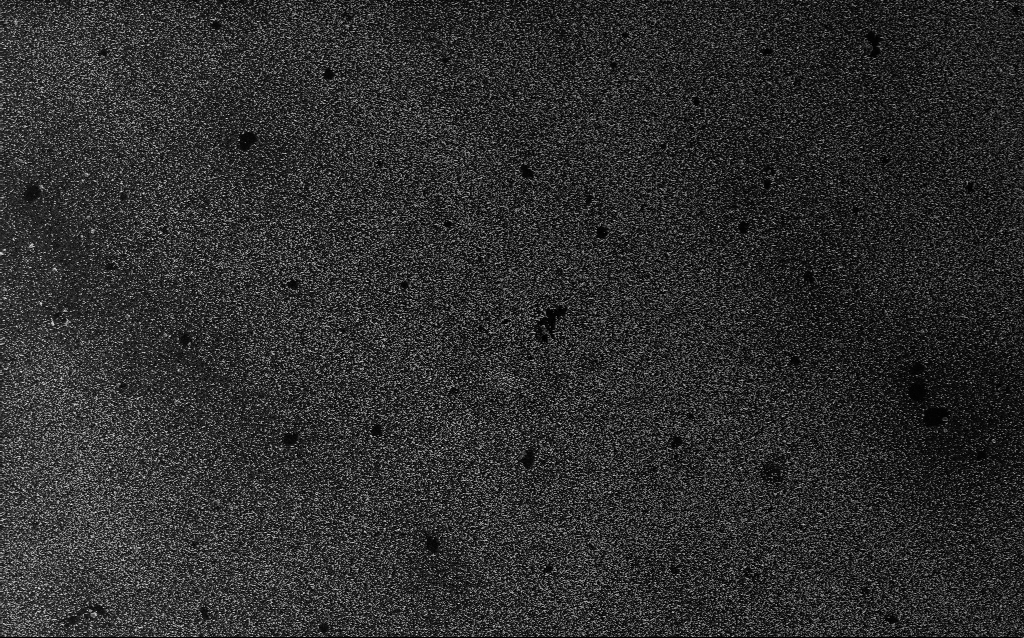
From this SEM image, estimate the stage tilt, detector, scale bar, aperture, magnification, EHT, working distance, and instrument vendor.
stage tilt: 0°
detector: InLens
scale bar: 10000 nm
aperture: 30 µm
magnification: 2 K X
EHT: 5 kV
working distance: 2.1 mm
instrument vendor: Zeiss